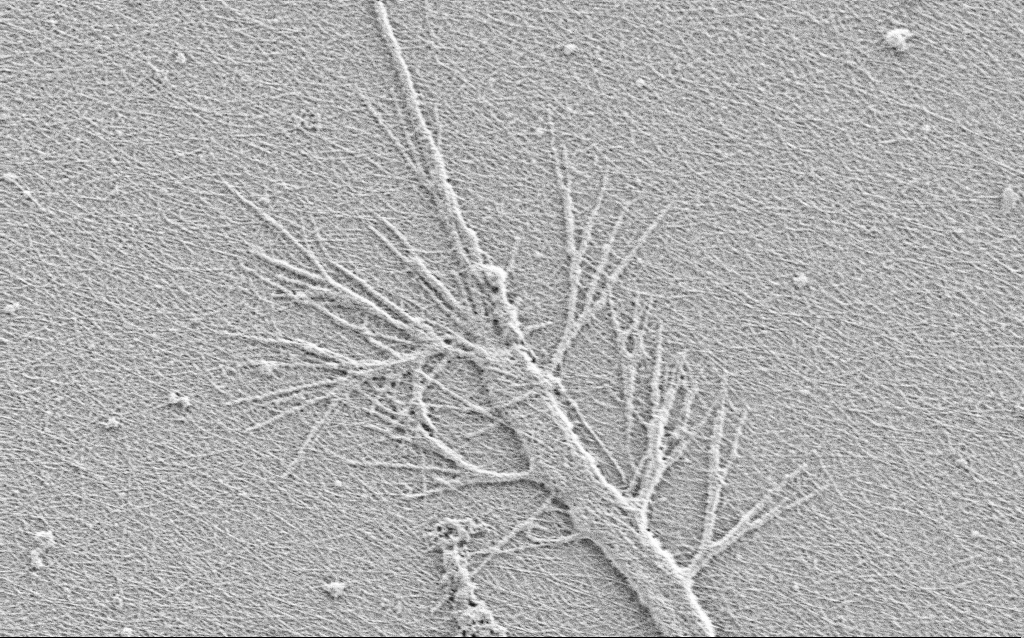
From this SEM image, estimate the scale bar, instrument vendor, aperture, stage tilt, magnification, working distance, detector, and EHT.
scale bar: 2000 nm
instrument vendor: Zeiss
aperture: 30 µm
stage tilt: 0°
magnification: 10 K X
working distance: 3 mm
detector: SE2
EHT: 0.9 kV